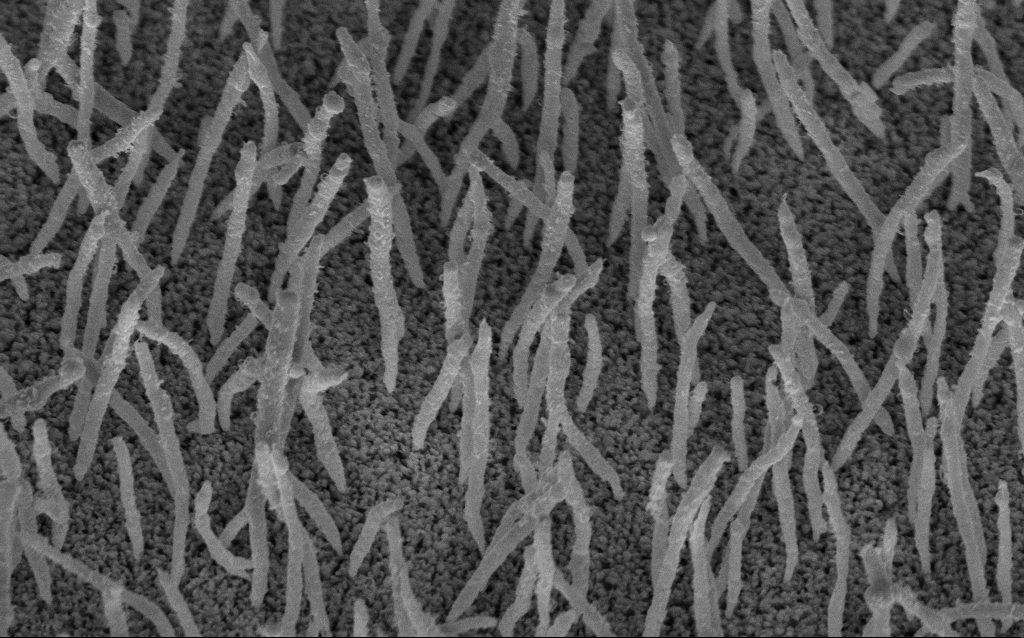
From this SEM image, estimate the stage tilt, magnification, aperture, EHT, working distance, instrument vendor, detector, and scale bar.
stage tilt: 45°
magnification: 50 K X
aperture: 30 µm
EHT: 5 kV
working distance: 8.2 mm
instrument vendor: Zeiss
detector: InLens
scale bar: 1000 nm